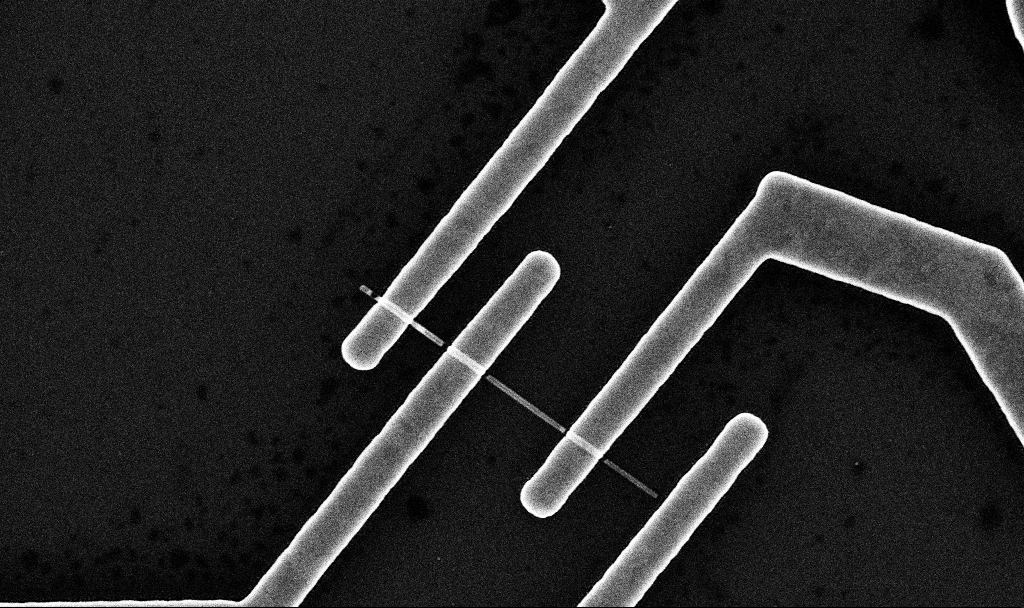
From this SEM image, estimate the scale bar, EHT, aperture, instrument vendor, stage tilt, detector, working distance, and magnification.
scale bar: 1000 nm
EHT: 10 kV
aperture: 30 µm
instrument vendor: Zeiss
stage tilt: -0°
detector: InLens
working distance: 7 mm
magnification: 36.58 K X